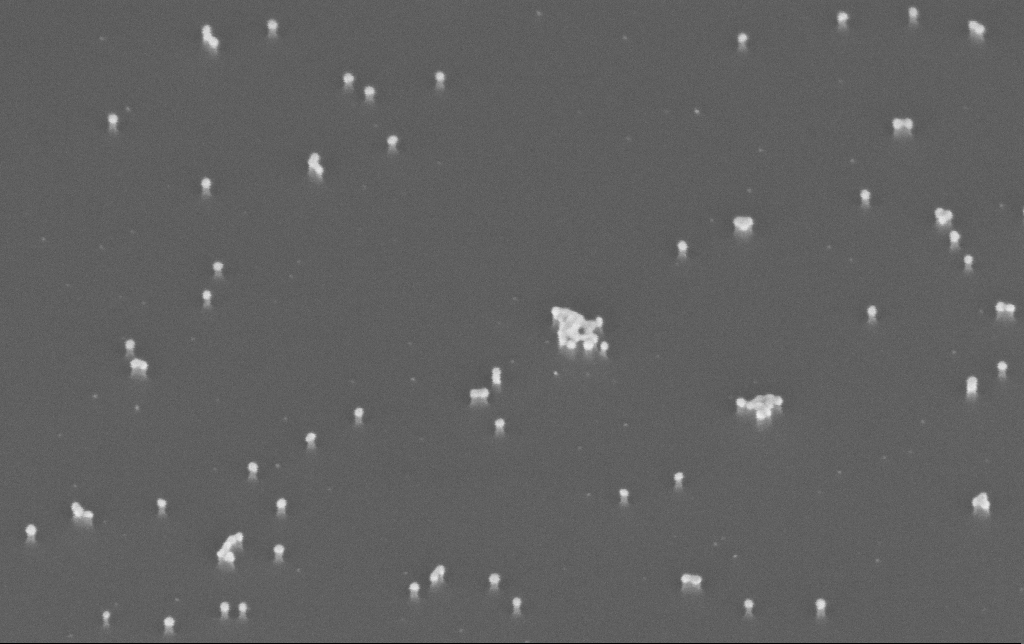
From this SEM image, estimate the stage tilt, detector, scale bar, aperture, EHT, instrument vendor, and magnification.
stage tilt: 45°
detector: InLens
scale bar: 100 nm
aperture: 30 µm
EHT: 10 kV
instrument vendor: Zeiss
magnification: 200 K X